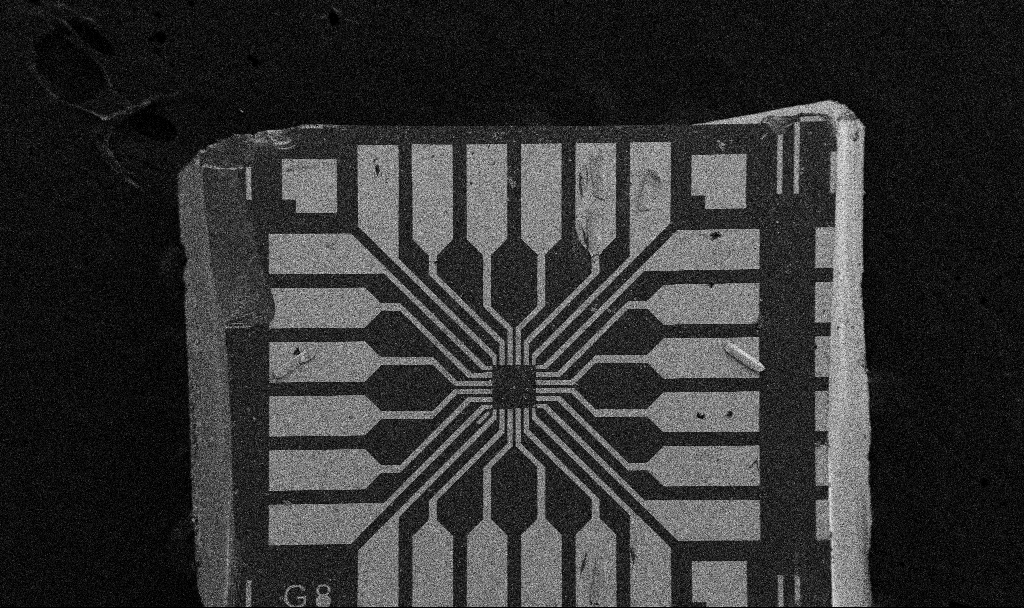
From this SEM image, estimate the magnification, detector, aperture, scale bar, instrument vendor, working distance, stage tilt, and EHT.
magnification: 0.1 K X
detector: SE2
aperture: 30 µm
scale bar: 200000 nm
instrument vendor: Zeiss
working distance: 8.5 mm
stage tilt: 0°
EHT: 5 kV